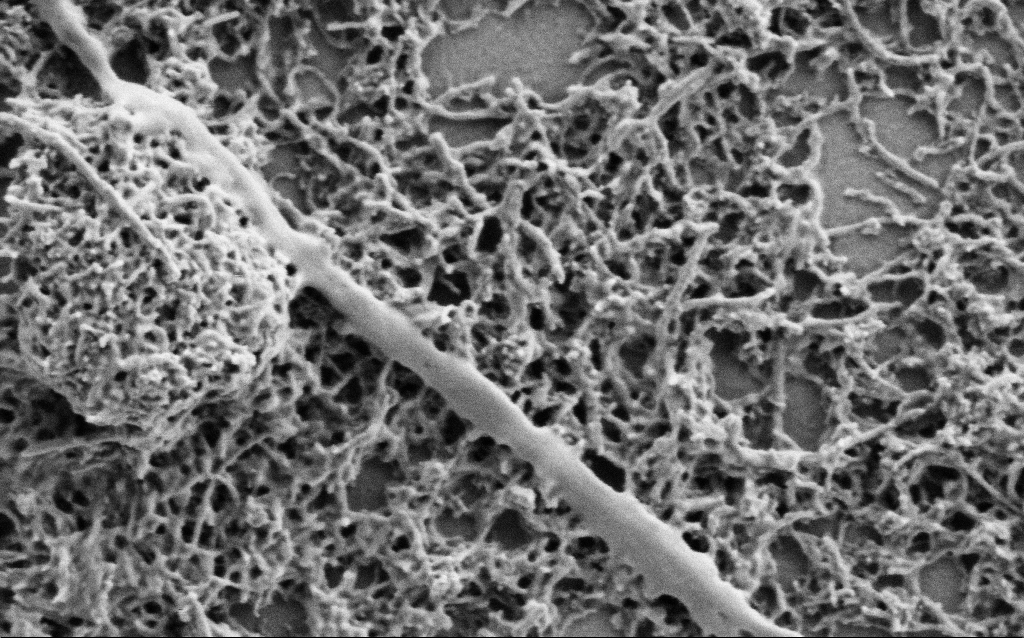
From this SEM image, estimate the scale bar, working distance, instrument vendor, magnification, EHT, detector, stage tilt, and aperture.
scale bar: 200 nm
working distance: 7 mm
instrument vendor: Zeiss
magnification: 75 K X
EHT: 1 kV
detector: SE2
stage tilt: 0°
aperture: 30 µm